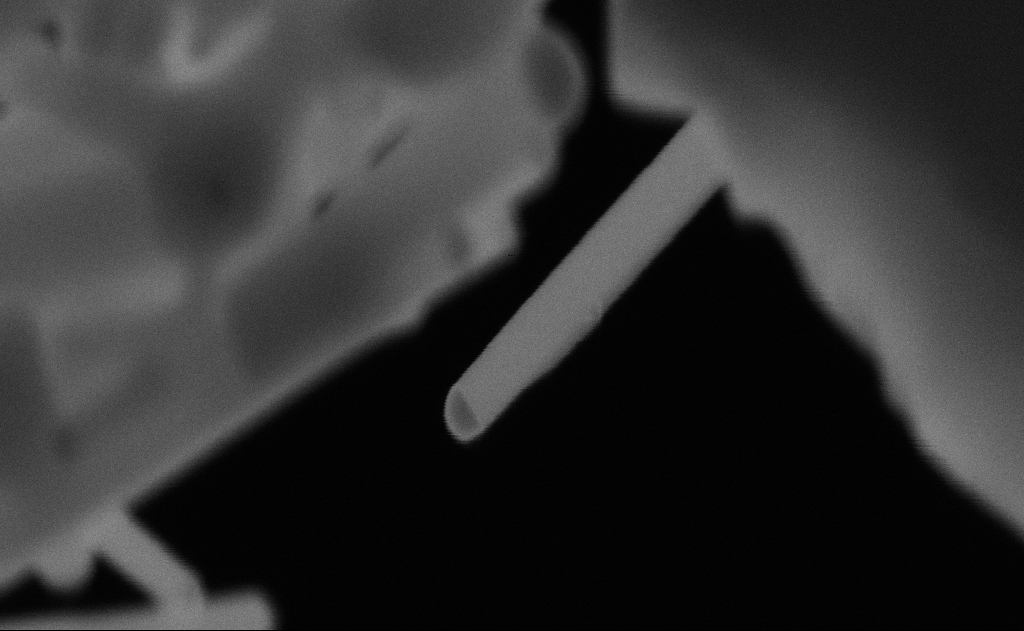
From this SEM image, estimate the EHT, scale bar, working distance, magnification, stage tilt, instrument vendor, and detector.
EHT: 20 kV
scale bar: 100 nm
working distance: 9 mm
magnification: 393.68 K X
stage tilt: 0°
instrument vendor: Zeiss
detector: SE2